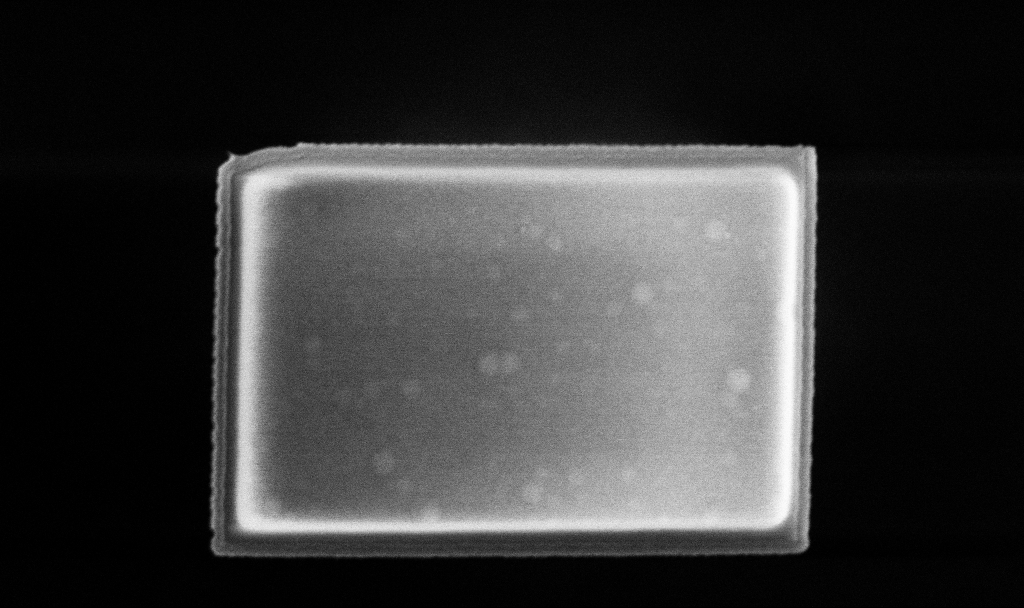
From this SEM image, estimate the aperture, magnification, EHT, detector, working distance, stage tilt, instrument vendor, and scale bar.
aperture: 30 µm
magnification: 51.2 K X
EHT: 5 kV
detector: InLens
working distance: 3.3 mm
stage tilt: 0°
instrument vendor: Zeiss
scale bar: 1000 nm